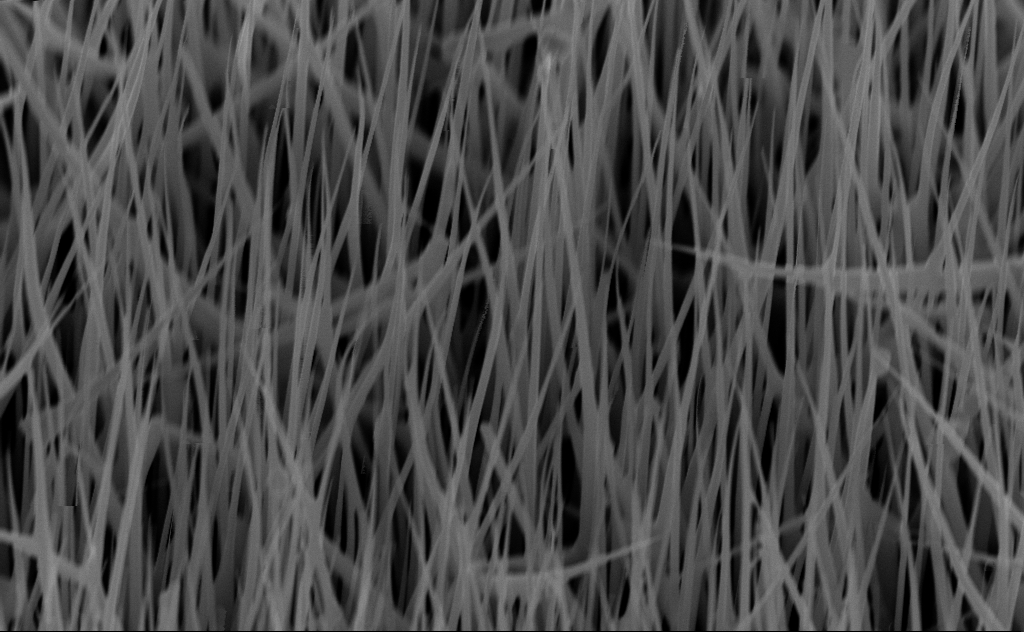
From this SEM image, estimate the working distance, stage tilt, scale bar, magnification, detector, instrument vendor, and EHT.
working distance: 7 mm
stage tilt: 45°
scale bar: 1000 nm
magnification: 40 K X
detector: InLens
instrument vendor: Zeiss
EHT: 10 kV